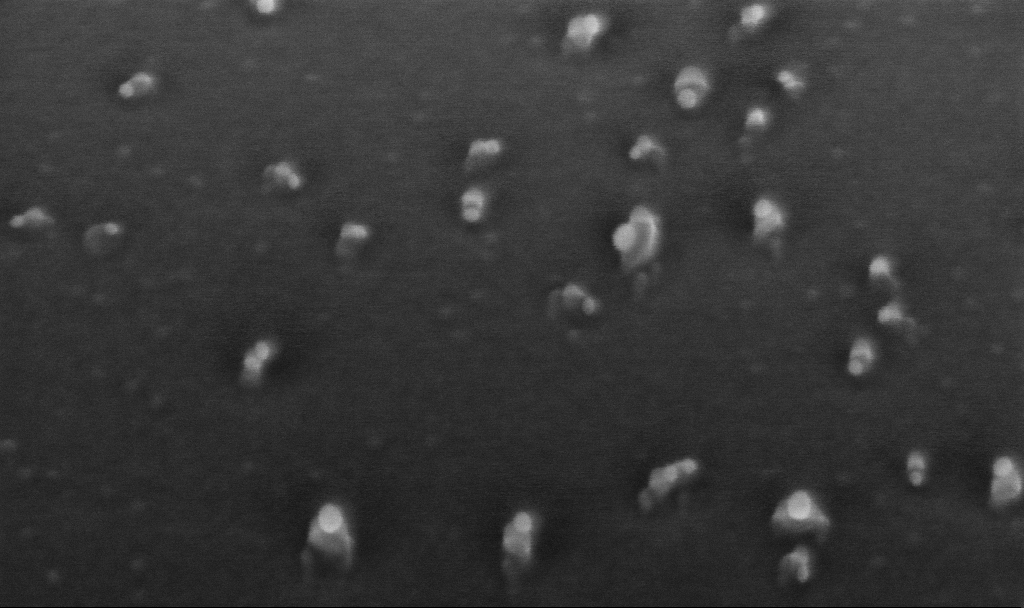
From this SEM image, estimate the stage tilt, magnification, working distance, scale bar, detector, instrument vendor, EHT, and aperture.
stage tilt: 45°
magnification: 300 K X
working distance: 4.4 mm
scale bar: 200 nm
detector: InLens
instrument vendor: Zeiss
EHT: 10 kV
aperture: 30 µm